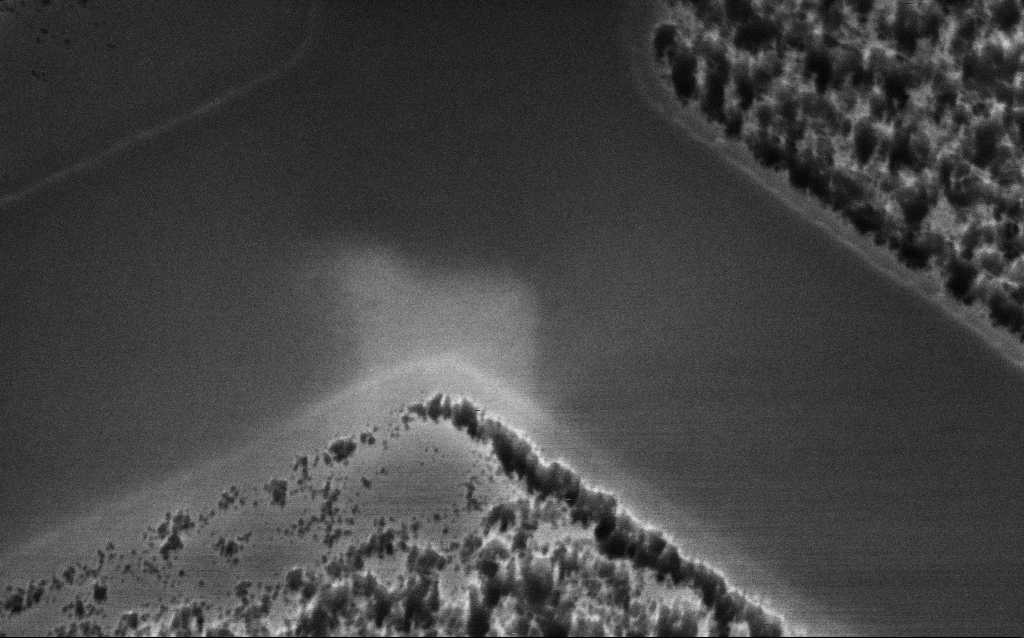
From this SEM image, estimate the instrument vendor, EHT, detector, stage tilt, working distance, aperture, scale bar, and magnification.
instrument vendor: Zeiss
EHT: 3 kV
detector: InLens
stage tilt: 45°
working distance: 8 mm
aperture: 30 µm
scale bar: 200 nm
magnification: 130.75 K X